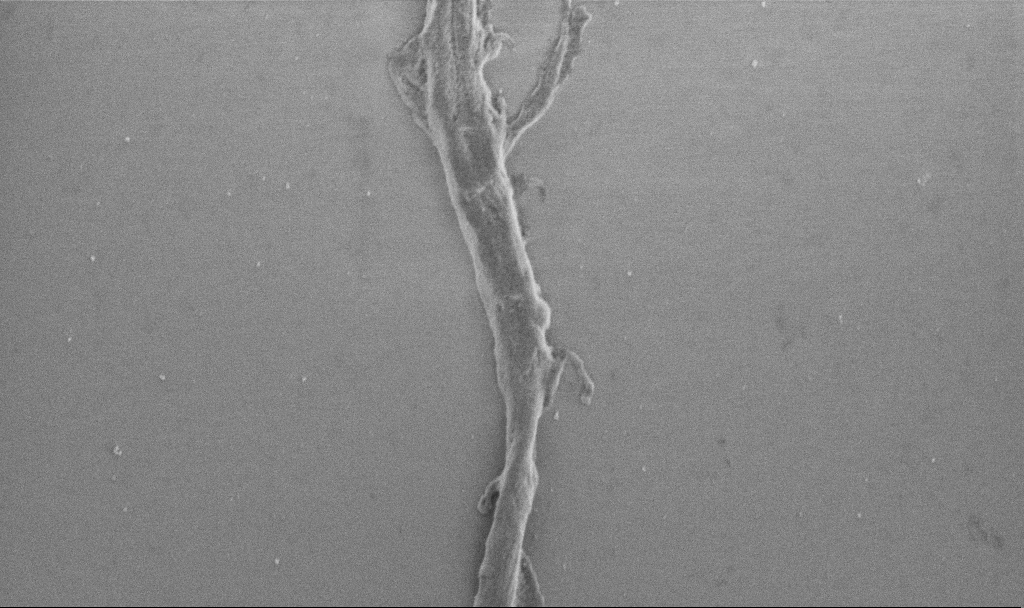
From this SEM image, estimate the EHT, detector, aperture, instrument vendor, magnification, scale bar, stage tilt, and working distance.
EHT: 1 kV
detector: SE2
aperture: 30 µm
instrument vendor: Zeiss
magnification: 20 K X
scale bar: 2000 nm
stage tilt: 0°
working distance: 6.9 mm